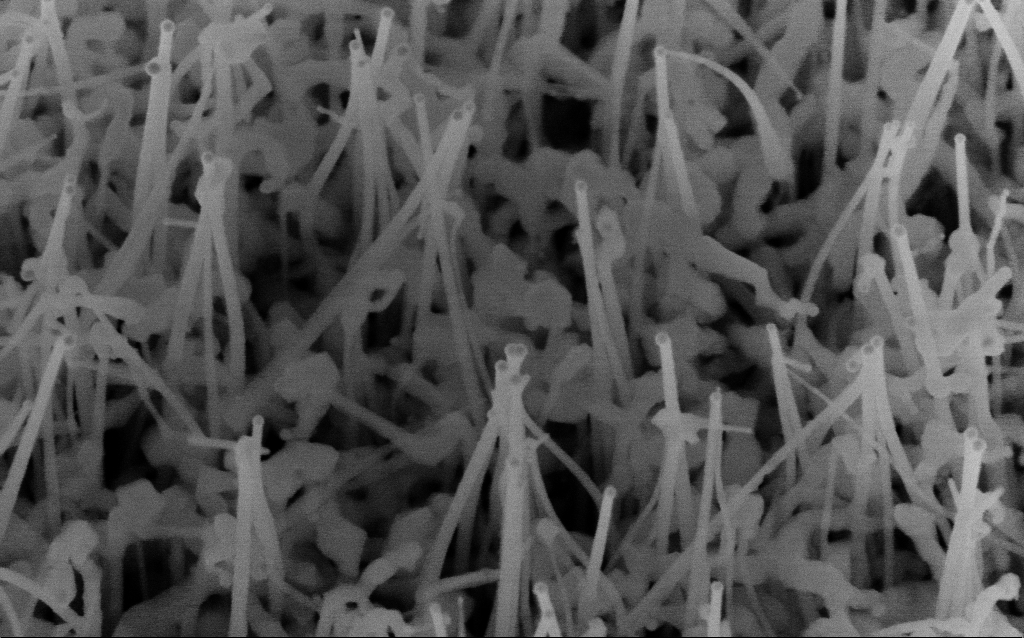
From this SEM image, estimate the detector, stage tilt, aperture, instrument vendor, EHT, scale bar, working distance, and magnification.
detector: InLens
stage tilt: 35°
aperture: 30 µm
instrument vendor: Zeiss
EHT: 10 kV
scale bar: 100 nm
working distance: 7.3 mm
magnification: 149.8 K X